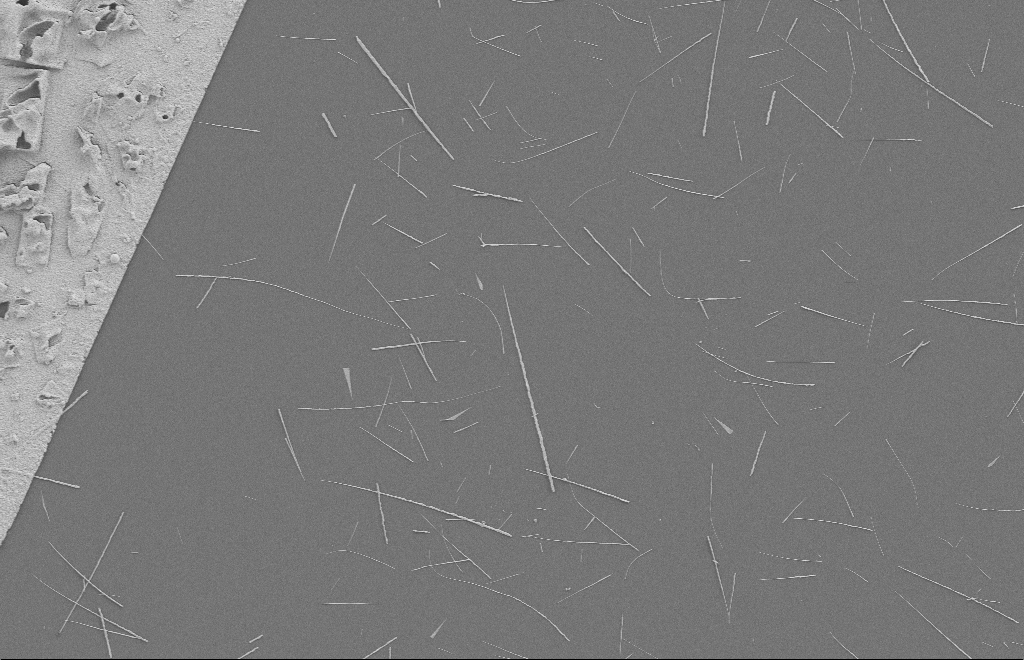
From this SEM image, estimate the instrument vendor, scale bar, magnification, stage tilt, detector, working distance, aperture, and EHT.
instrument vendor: Zeiss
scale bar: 10000 nm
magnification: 4.54 K X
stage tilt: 0°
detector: SE2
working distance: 8 mm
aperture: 20 µm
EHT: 2 kV